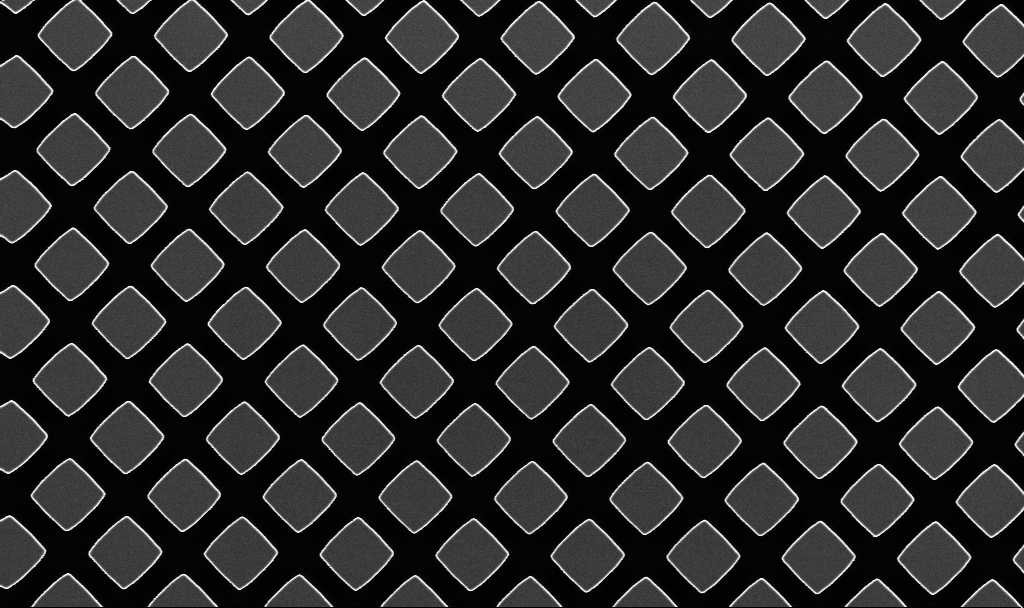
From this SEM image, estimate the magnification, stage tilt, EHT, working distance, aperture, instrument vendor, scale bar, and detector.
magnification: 4.99 K X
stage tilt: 0°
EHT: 3 kV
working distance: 7.7 mm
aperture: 30 µm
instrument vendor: Zeiss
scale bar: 10000 nm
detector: InLens